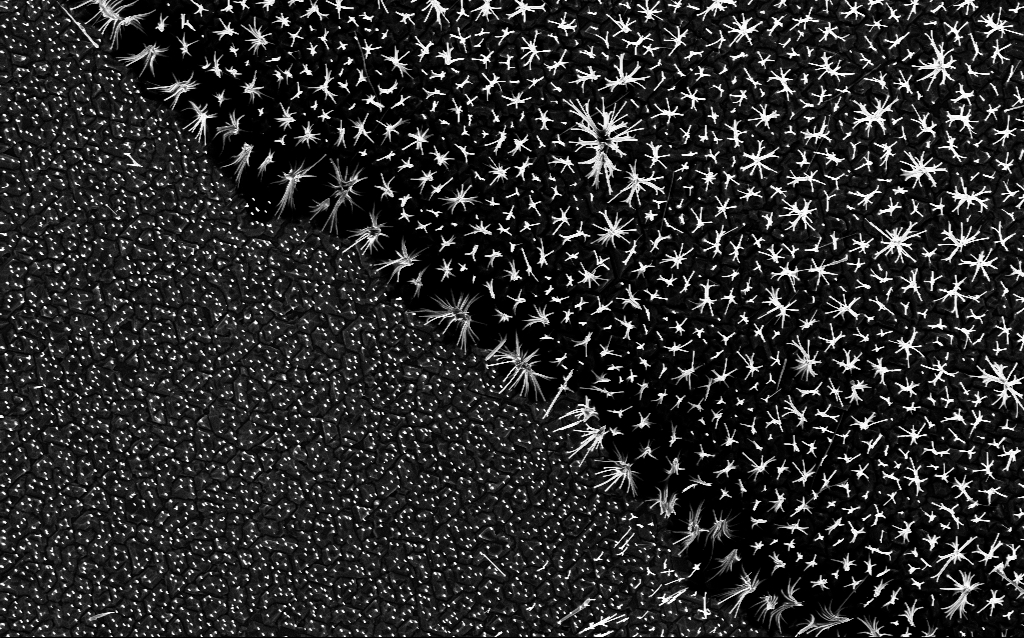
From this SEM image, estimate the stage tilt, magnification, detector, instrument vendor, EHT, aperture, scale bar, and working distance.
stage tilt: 0°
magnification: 6.78 K X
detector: InLens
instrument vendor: Zeiss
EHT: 5 kV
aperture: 30 µm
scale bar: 10000 nm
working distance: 6.6 mm